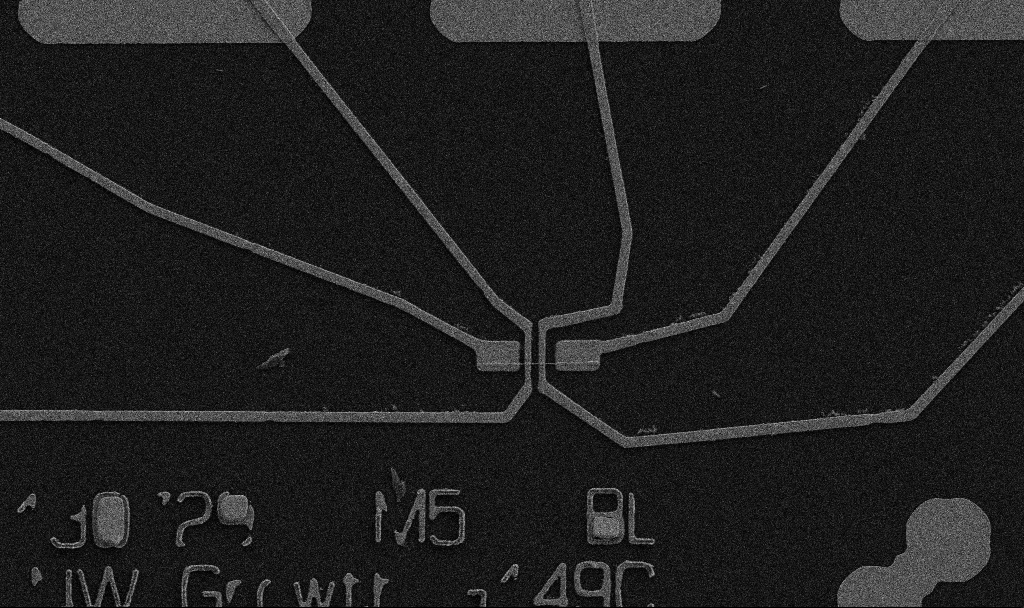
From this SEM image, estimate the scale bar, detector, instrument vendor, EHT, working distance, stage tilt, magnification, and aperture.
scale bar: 10000 nm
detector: SE2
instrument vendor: Zeiss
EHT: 5 kV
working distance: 10.7 mm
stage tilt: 0°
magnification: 5 K X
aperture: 30 µm